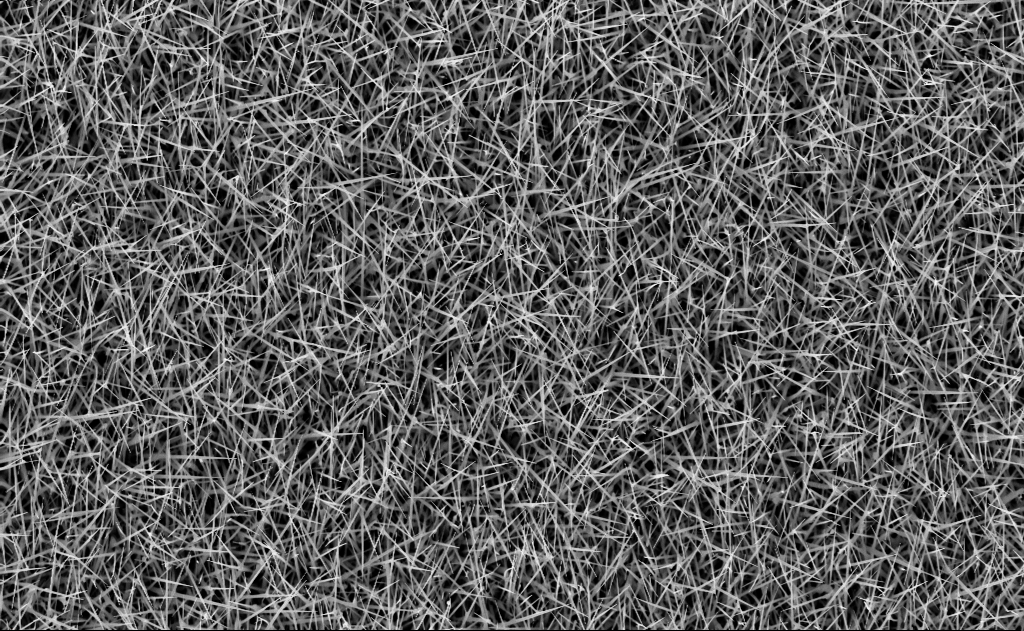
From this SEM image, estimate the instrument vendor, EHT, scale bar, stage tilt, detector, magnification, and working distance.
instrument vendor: Zeiss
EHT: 10 kV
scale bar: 2000 nm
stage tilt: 0°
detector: InLens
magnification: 10 K X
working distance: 7 mm